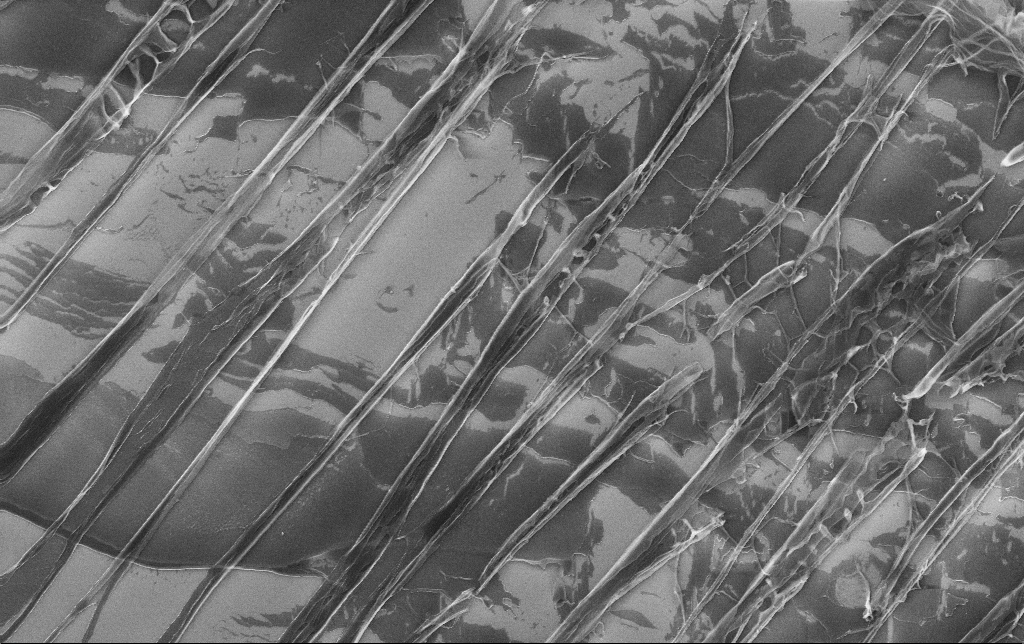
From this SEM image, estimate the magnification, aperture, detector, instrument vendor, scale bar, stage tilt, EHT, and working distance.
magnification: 6.07 K X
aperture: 30 µm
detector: InLens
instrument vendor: Zeiss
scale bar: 10000 nm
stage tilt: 0°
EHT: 10 kV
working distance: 3.1 mm